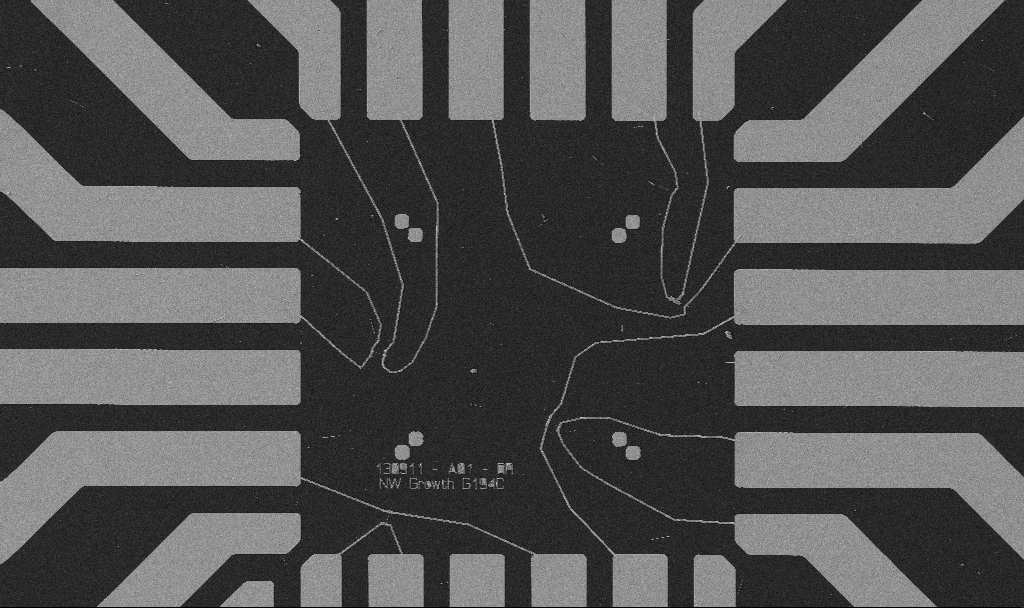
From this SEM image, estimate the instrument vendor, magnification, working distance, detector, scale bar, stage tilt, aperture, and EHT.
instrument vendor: Zeiss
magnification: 1 K X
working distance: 10.7 mm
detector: SE2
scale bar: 20000 nm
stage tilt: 0°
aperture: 30 µm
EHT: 5 kV